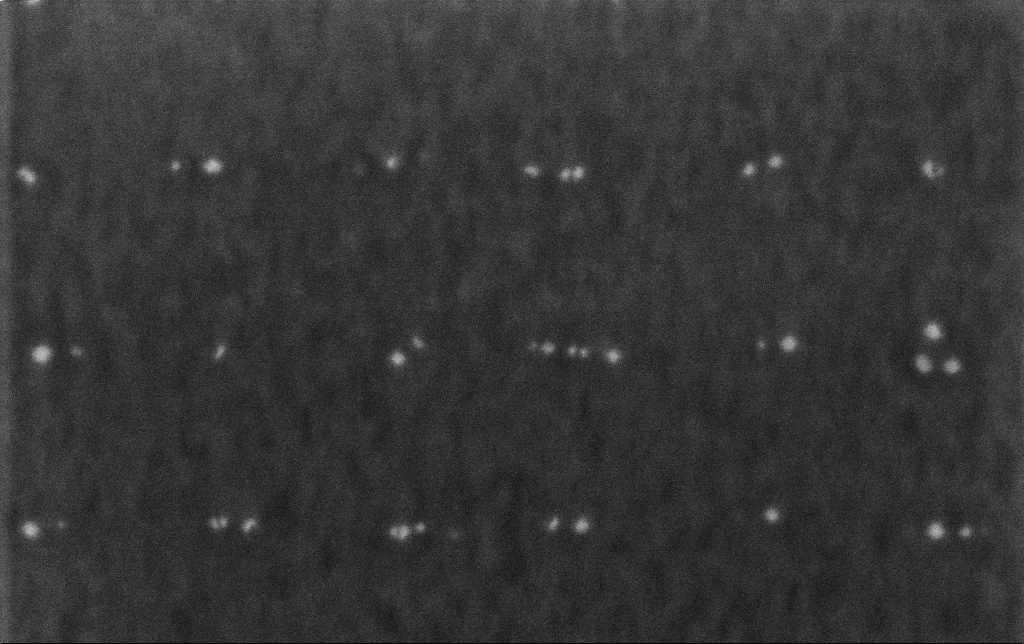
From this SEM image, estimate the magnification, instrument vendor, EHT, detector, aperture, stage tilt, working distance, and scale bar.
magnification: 219.35 K X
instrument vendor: Zeiss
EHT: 3 kV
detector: InLens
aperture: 30 µm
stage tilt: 0°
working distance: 3.2 mm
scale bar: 200 nm